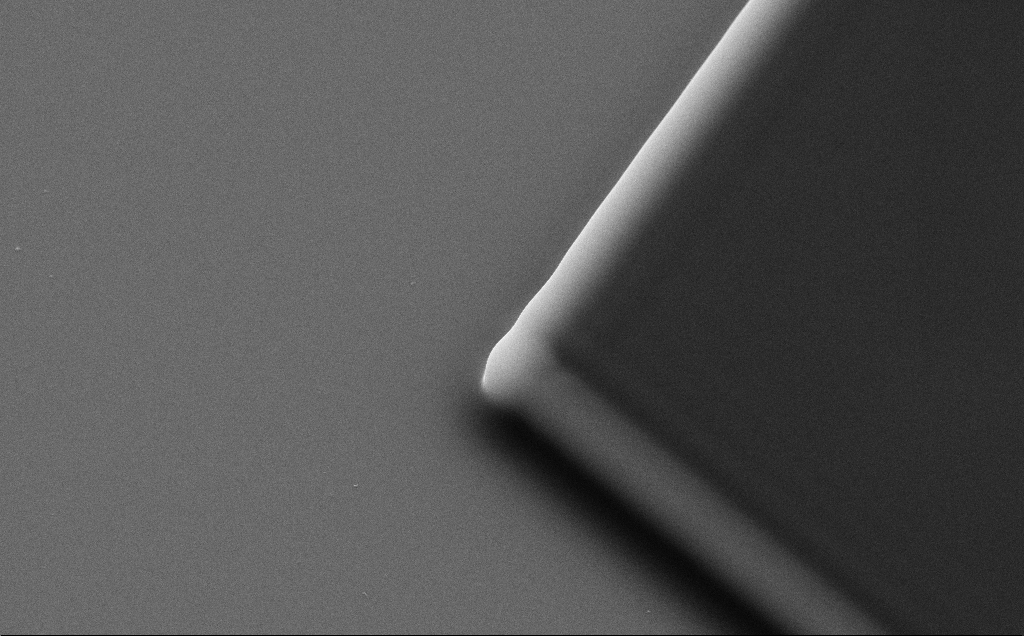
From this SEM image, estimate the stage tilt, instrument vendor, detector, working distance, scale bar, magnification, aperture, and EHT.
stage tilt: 35°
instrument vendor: Zeiss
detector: SE2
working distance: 8 mm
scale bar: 2000 nm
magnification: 16.47 K X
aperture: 30 µm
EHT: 10 kV